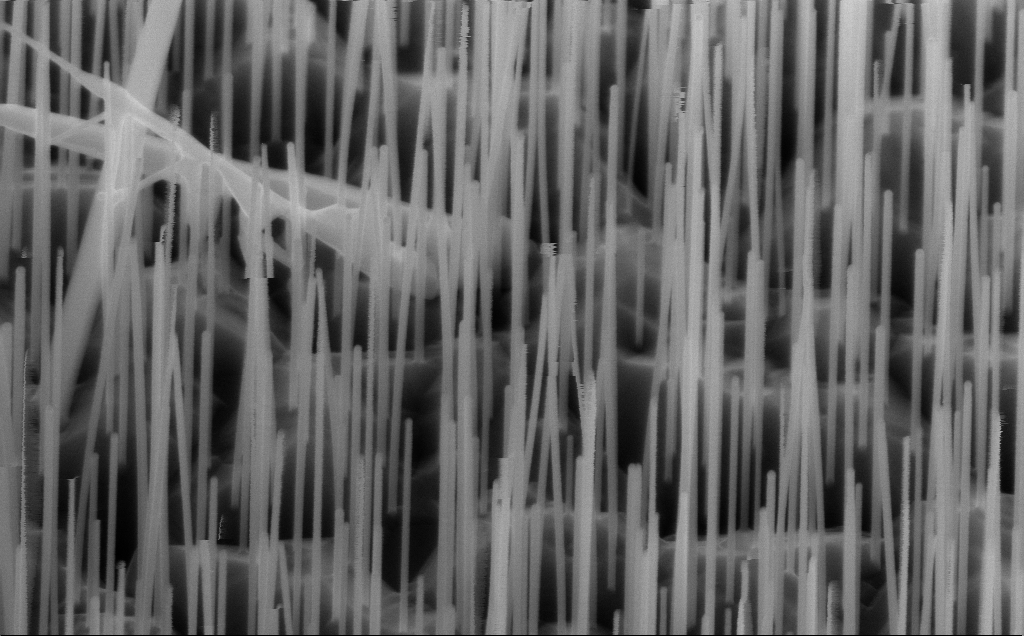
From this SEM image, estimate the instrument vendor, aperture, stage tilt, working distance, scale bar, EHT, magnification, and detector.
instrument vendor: Zeiss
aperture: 30 µm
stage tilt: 45°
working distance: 6 mm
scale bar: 200 nm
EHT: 10 kV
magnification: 80 K X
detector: InLens